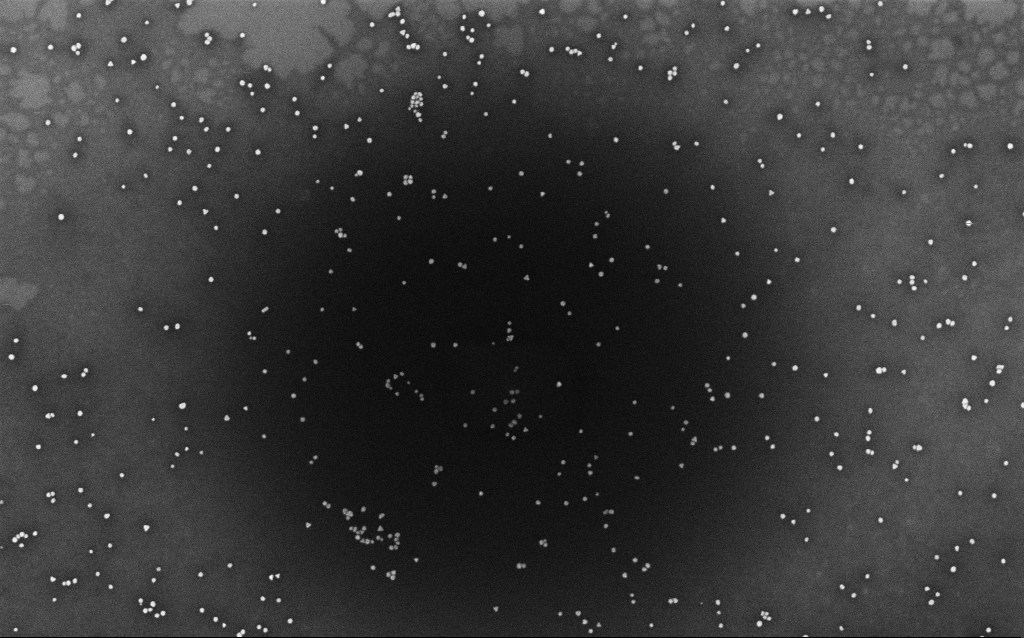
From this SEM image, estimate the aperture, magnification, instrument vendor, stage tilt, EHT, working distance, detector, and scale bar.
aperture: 30 µm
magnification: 100 K X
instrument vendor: Zeiss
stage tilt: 0°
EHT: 10 kV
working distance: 1.9 mm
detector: InLens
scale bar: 200 nm